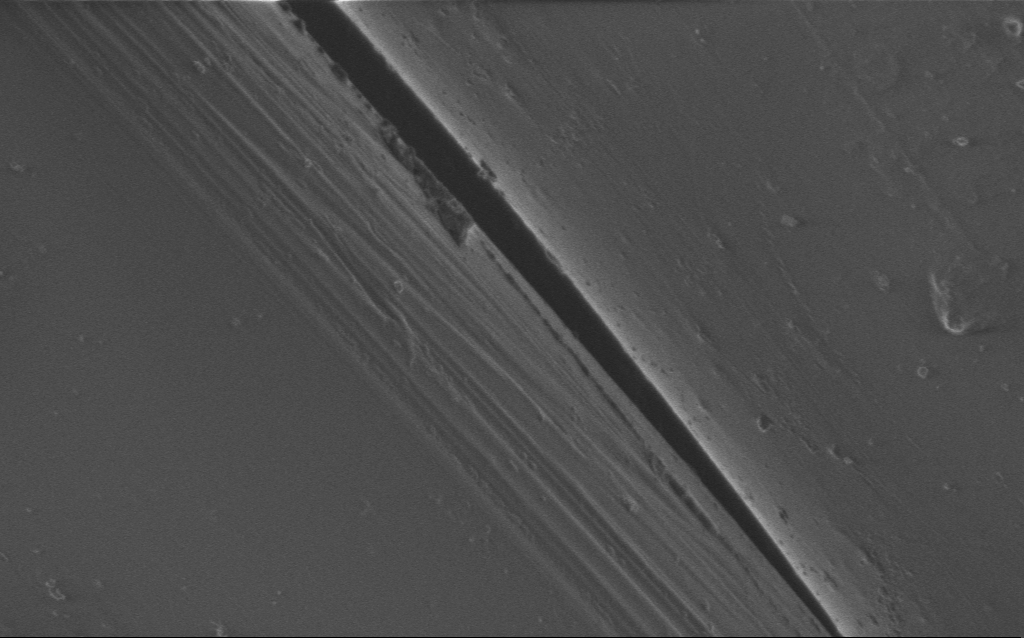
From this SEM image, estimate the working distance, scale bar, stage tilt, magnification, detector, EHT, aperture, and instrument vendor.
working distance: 4 mm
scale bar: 2000 nm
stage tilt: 0°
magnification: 11.58 K X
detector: InLens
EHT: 1 kV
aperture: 30 µm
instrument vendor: Zeiss